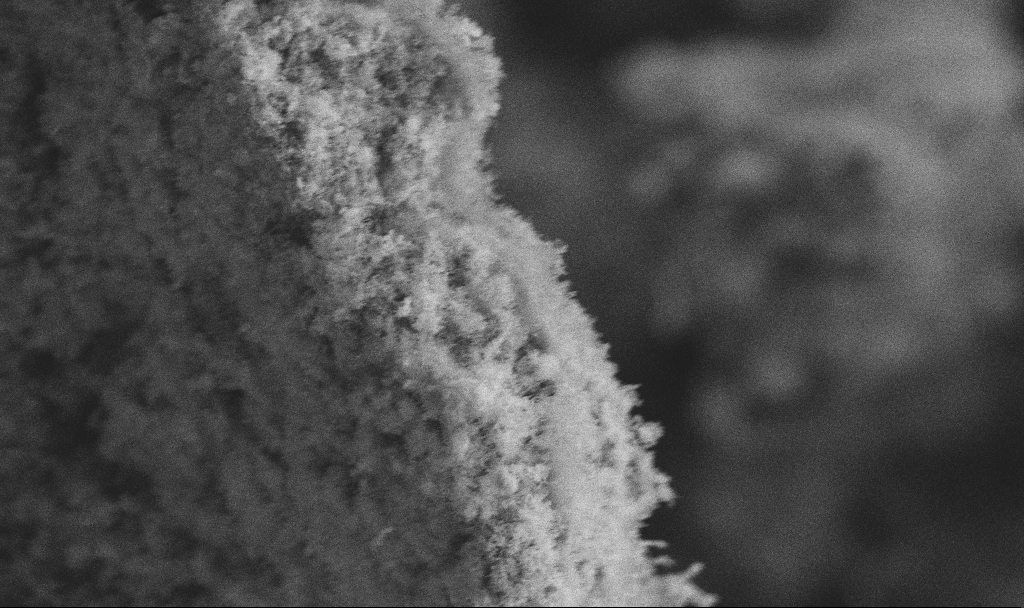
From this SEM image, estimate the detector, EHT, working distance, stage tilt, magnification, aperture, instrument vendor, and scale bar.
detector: SE2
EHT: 3 kV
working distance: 3.8 mm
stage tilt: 0°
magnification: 25 K X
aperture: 30 µm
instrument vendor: Zeiss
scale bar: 1000 nm